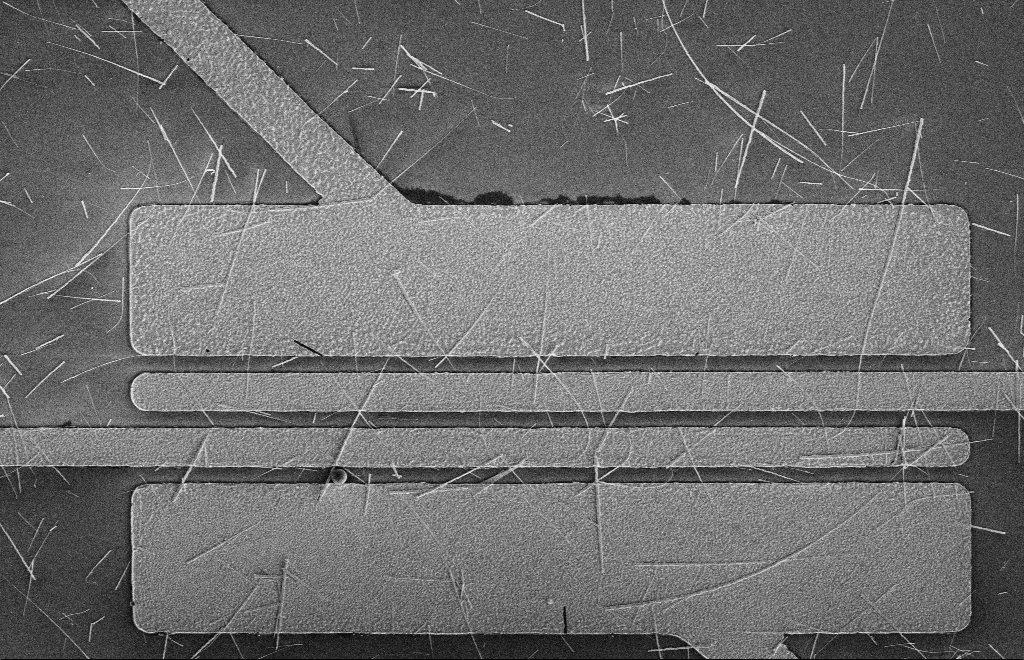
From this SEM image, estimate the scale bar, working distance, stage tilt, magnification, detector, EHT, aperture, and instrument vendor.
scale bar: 2000 nm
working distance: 16 mm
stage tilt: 0°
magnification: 5.06 K X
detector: SE2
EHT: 5 kV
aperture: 10 µm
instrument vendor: Zeiss